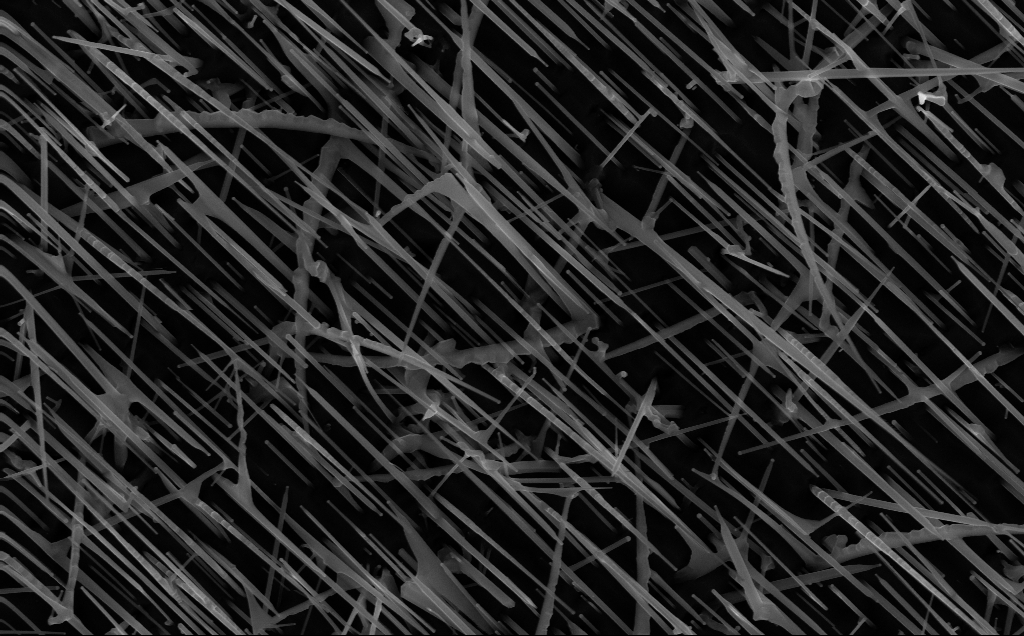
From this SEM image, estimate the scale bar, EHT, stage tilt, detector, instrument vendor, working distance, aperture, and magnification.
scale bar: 1000 nm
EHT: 10 kV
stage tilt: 0°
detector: InLens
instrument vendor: Zeiss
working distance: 5 mm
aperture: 30 µm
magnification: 40 K X